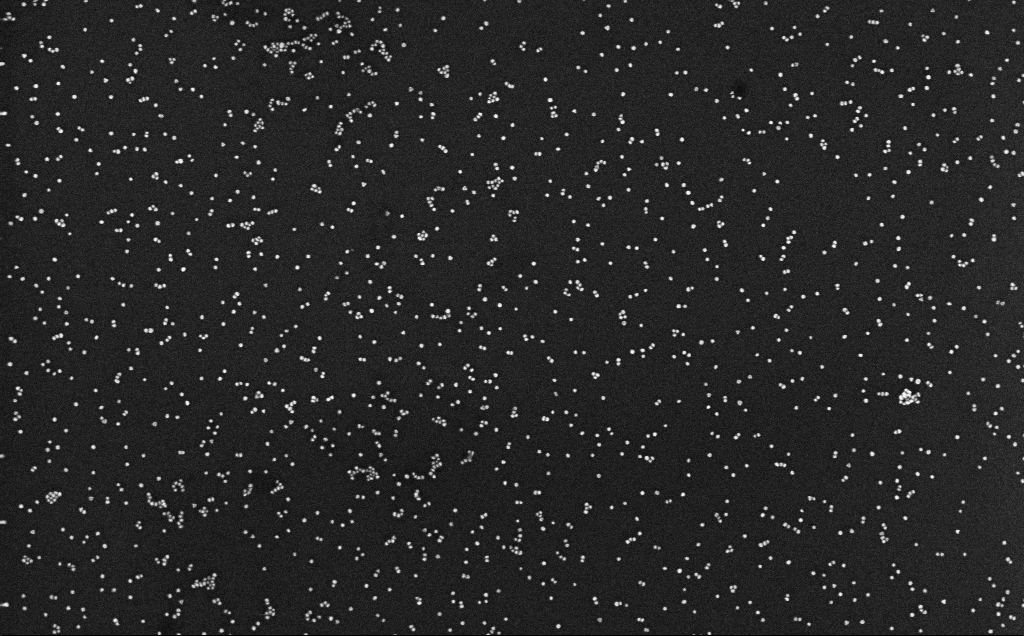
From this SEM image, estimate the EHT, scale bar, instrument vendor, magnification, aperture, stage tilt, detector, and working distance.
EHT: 10 kV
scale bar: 200 nm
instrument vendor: Zeiss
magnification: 100 K X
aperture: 30 µm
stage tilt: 0°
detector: InLens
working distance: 3.1 mm